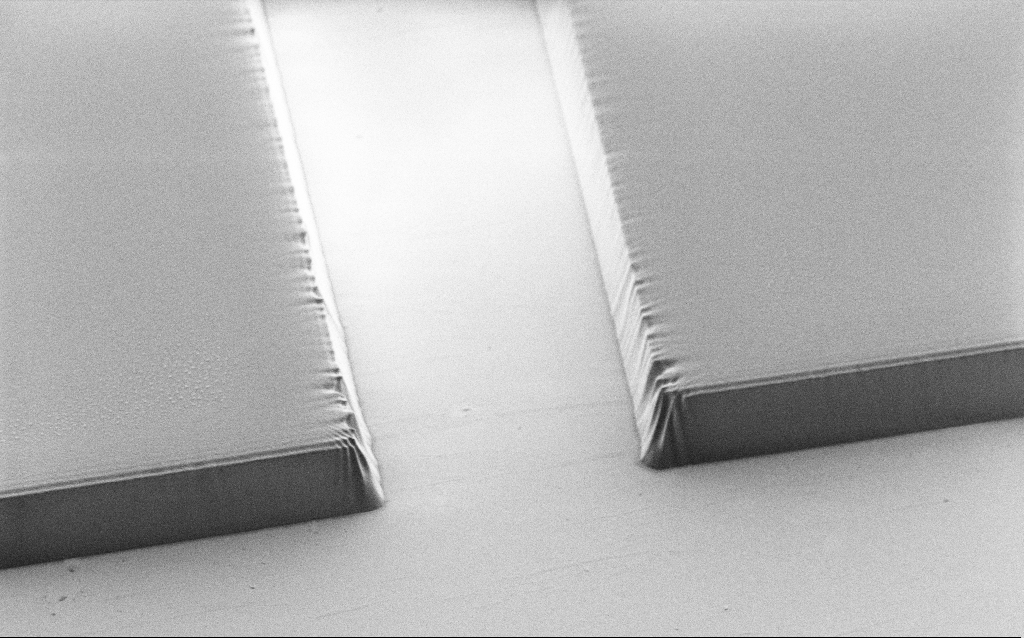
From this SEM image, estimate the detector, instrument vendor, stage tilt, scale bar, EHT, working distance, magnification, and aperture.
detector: SE2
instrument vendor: Zeiss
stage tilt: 45°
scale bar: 10000 nm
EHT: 1.2 kV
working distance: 8 mm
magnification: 4.74 K X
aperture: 30 µm